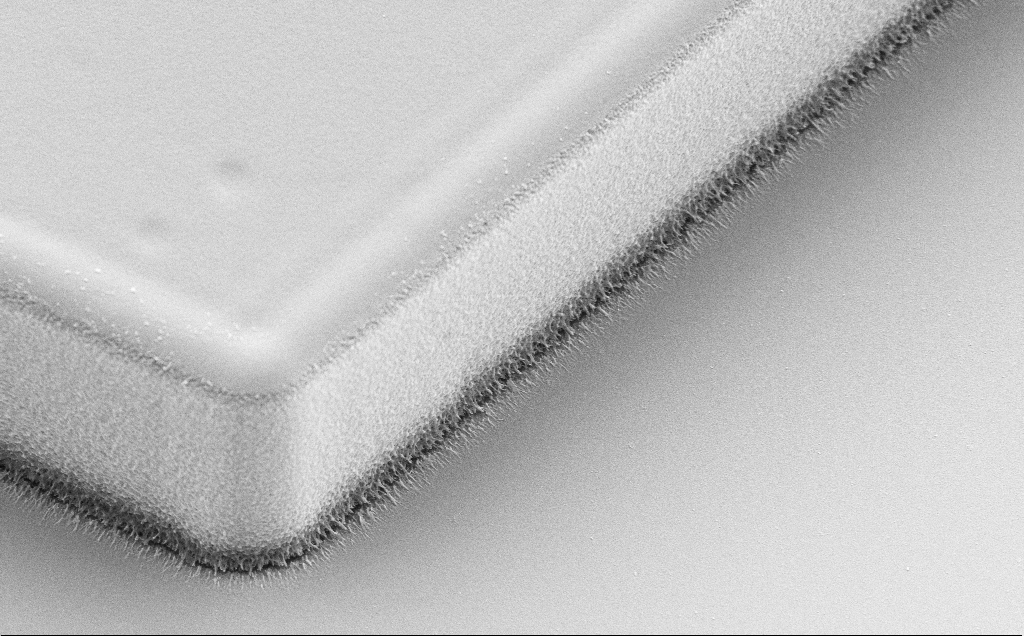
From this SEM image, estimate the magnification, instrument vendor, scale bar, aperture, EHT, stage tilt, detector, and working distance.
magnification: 12.94 K X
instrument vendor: Zeiss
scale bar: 1000 nm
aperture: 30 µm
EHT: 5 kV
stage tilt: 30°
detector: SE2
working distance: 8 mm